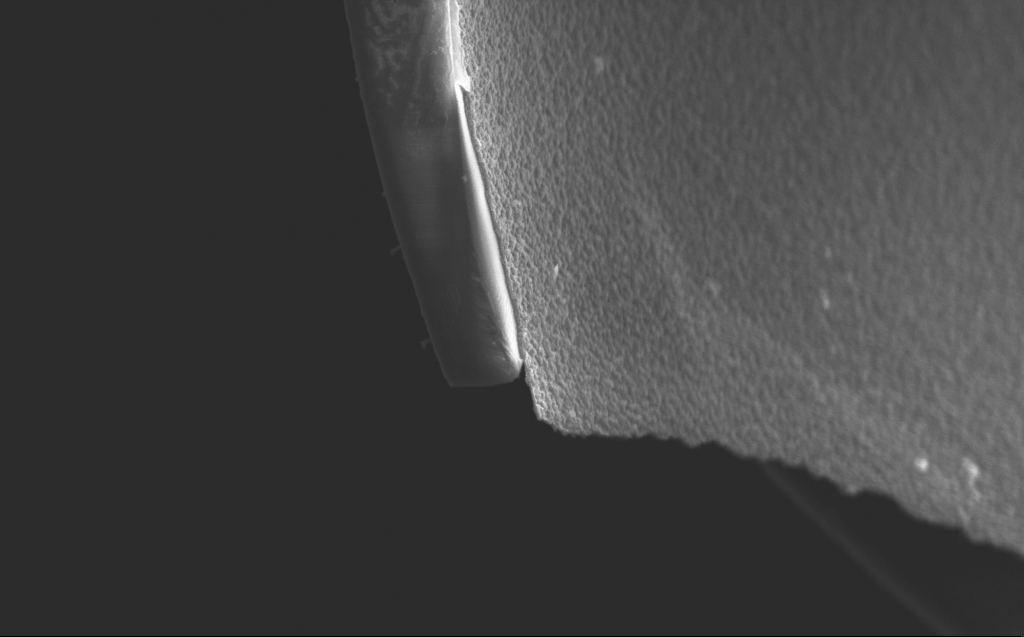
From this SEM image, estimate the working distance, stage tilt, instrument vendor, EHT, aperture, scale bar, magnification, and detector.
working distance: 4 mm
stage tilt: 45°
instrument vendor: Zeiss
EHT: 5 kV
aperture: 30 µm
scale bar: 2000 nm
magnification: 31.8 K X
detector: InLens